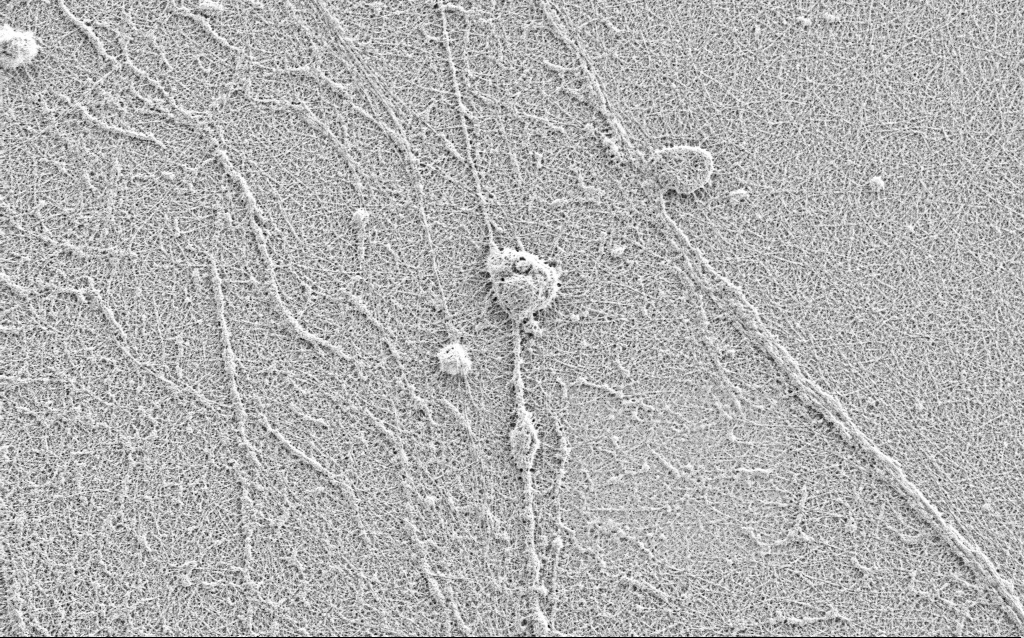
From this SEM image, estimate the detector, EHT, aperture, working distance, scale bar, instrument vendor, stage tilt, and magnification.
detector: SE2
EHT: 0.9 kV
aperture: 30 µm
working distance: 3 mm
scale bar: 2000 nm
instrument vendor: Zeiss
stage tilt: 0°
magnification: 10 K X